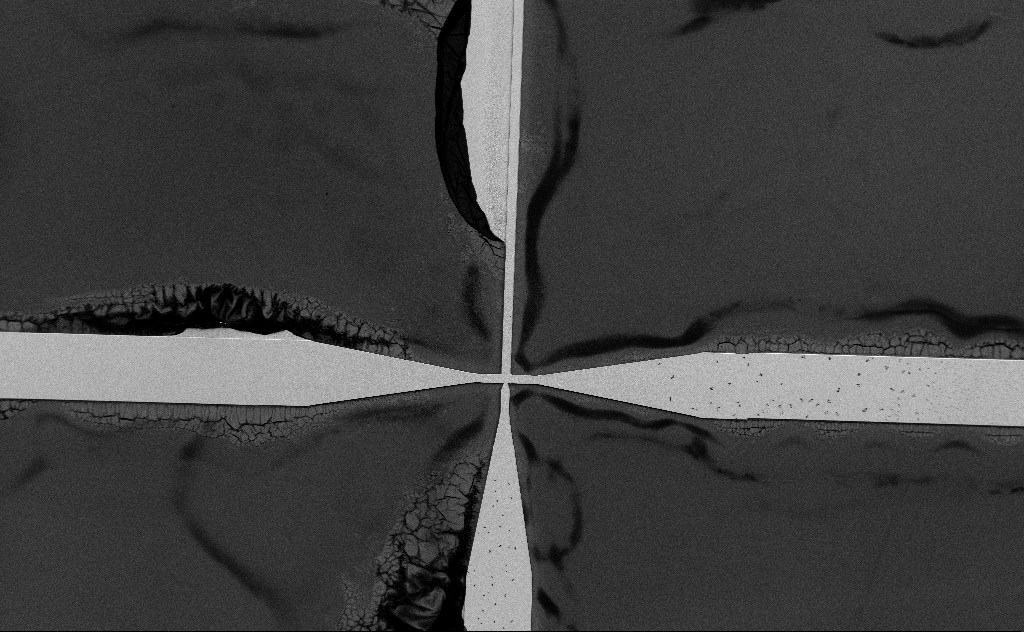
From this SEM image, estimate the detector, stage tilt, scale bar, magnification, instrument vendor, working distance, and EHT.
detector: SE2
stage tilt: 0°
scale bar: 100000 nm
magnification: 0.295 K X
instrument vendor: Zeiss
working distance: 14 mm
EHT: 3 kV